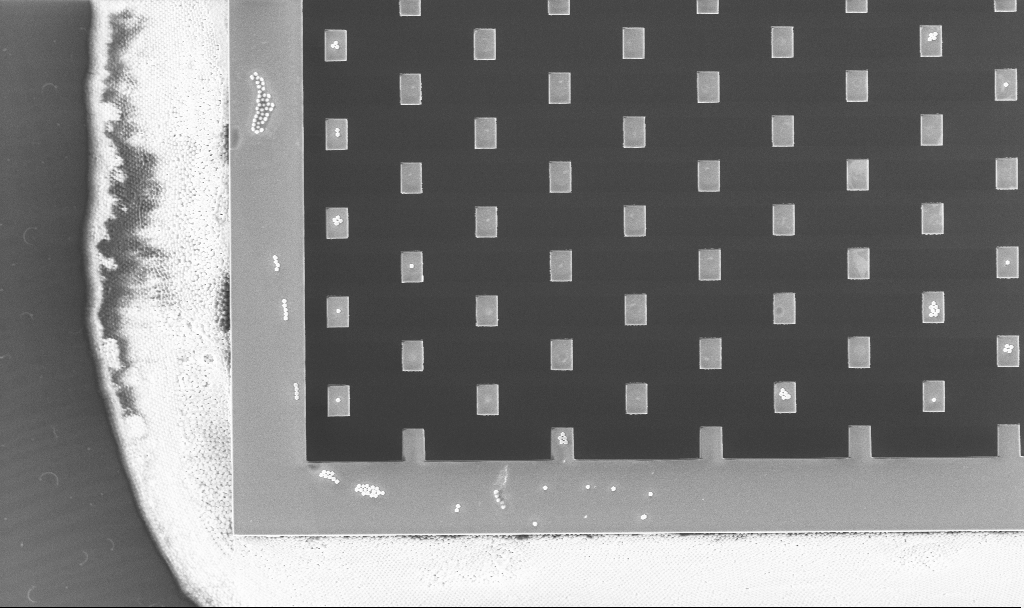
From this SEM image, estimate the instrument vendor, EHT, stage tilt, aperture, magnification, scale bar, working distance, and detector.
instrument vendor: Zeiss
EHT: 5 kV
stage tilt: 0°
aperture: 30 µm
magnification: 2.73 K X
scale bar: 10000 nm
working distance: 3.2 mm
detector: InLens